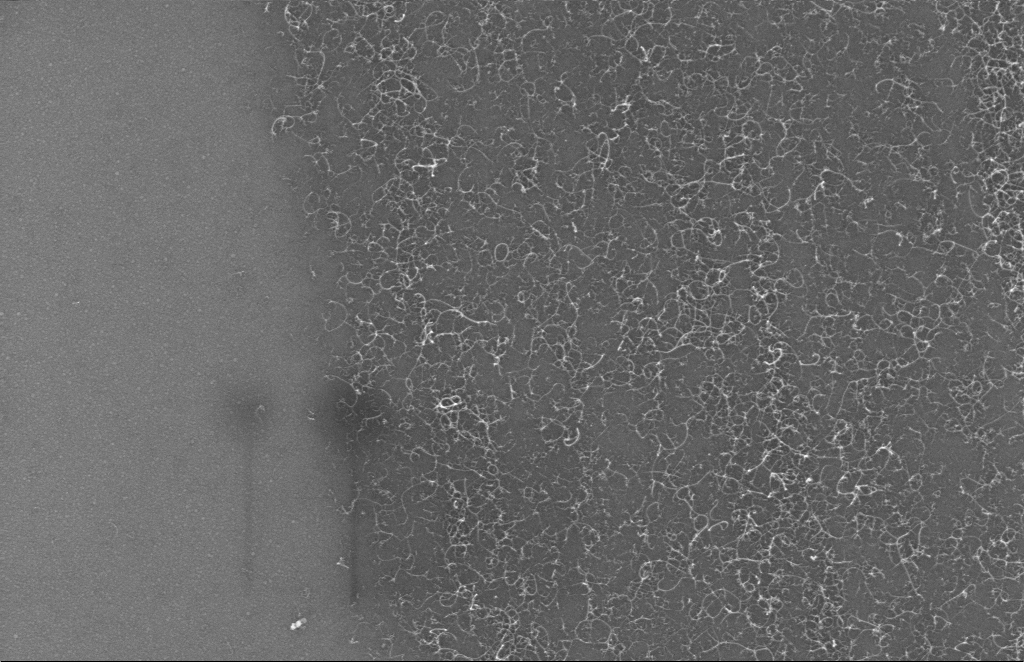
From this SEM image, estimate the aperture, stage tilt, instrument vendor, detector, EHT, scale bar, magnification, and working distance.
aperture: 30 µm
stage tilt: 0°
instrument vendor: Zeiss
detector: InLens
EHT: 5 kV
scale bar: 200 nm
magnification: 54.27 K X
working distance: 5 mm